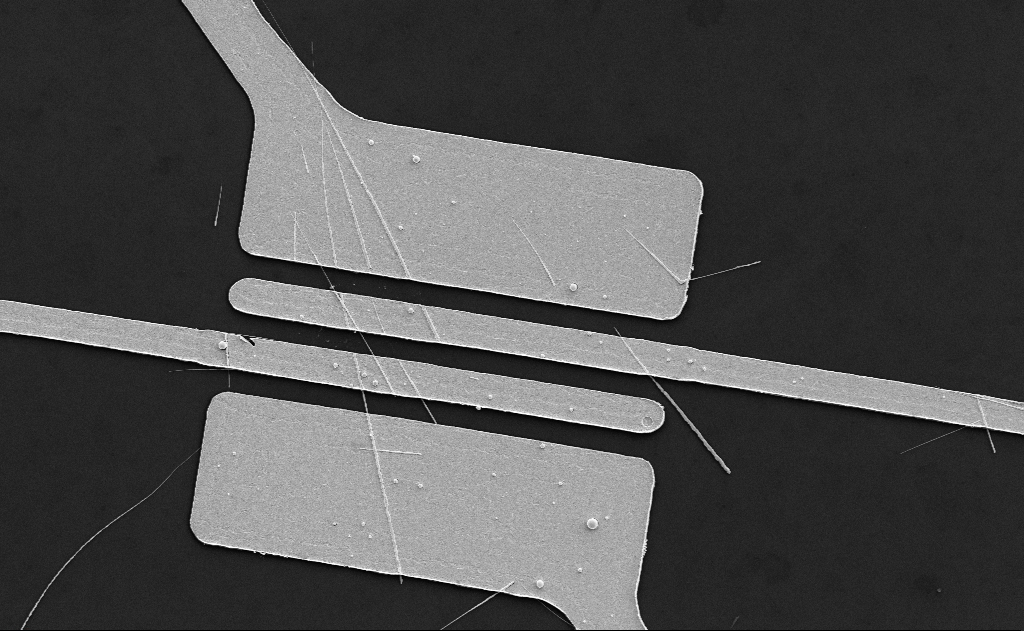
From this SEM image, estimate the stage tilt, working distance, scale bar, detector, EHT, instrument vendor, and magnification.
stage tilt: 0°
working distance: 19 mm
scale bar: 10000 nm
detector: SE2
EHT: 5 kV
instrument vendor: Zeiss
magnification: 5.43 K X